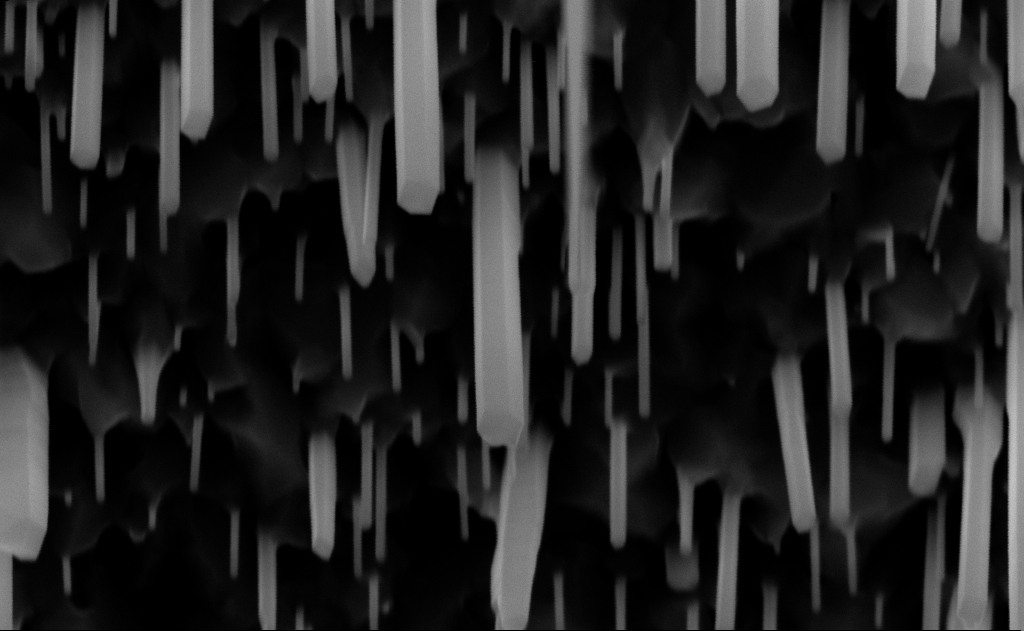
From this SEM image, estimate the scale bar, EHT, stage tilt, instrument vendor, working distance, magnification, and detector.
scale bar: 200 nm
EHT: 10 kV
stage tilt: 0°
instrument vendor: Zeiss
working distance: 9 mm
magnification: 100 K X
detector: InLens